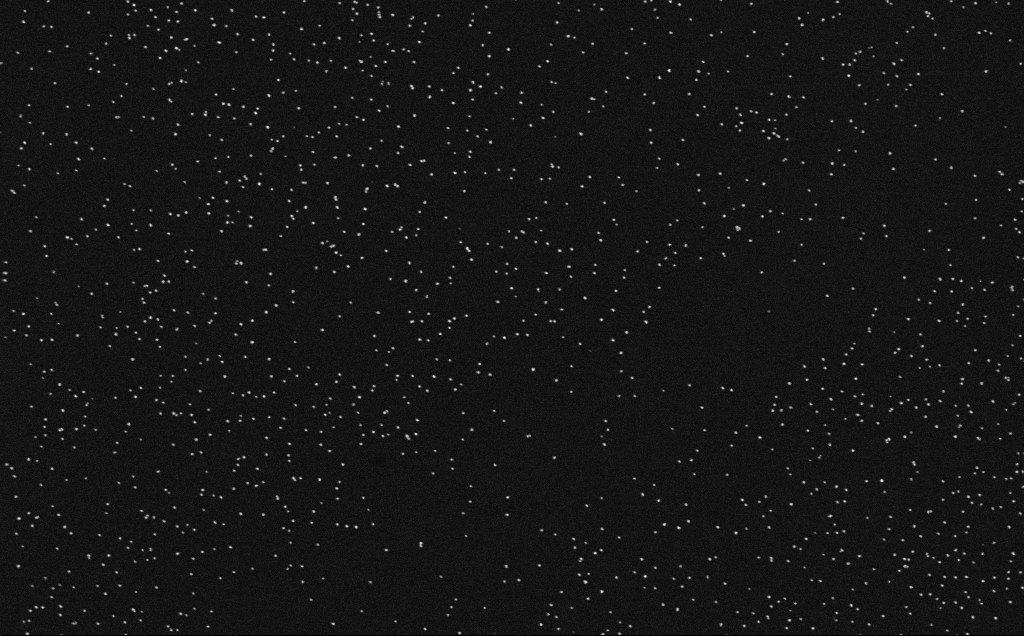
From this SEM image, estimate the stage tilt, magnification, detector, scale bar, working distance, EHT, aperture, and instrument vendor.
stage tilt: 0°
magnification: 100 K X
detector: InLens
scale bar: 200 nm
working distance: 4 mm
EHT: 10 kV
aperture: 30 µm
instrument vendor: Zeiss